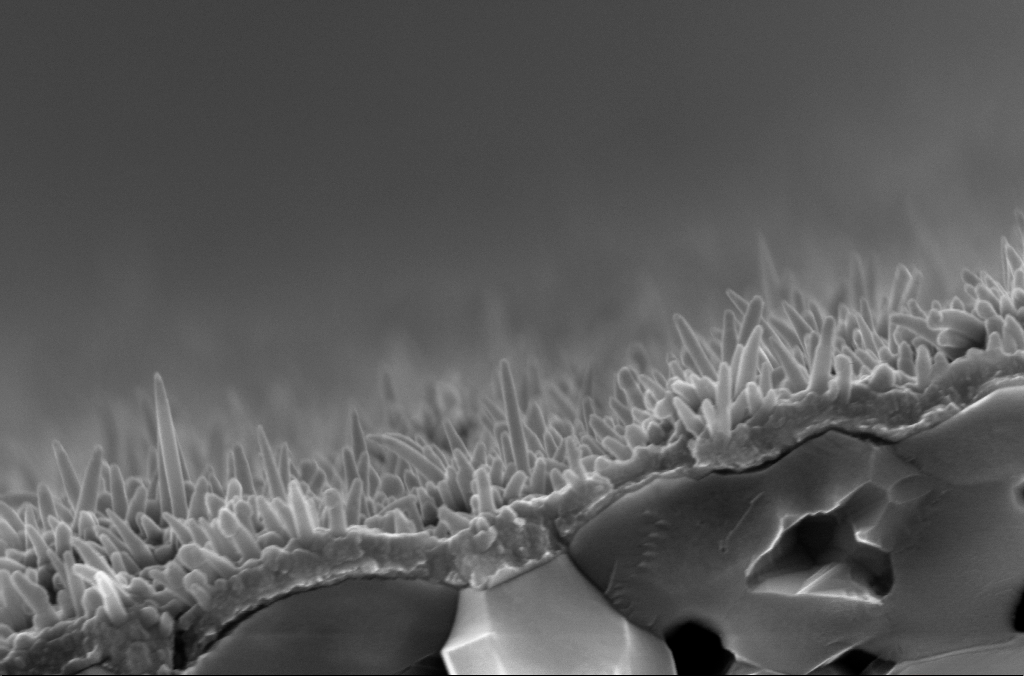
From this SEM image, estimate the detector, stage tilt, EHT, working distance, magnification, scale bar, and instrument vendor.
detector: InLens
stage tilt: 4°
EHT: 10 kV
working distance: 2.6 mm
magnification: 97.08 K X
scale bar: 200 nm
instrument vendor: Zeiss